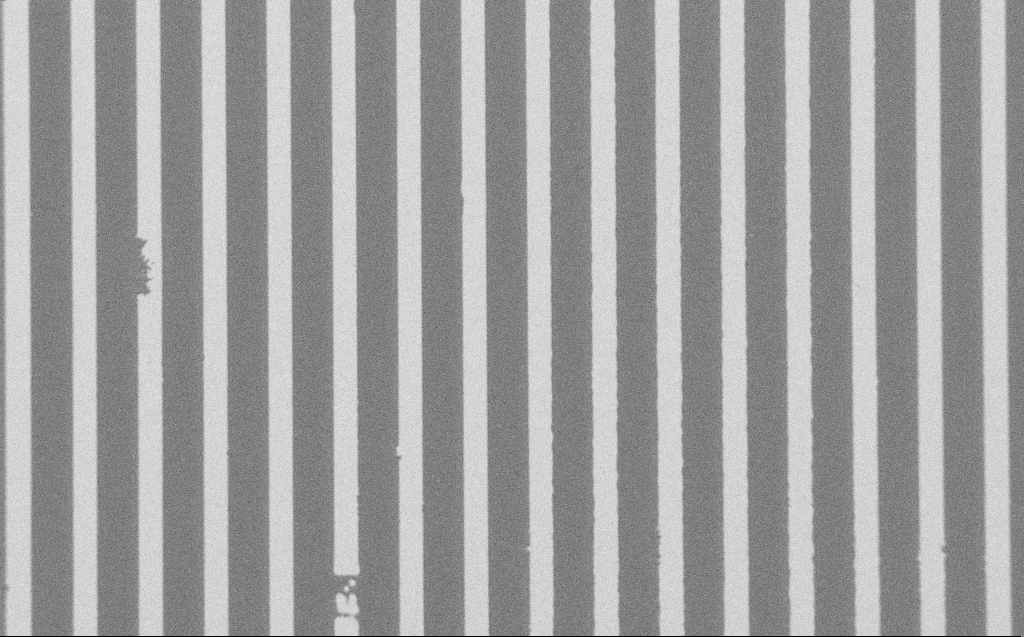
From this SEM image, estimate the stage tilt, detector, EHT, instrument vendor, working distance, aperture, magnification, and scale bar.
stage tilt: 0°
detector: SE2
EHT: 1.2 kV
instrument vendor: Zeiss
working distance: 6 mm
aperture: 30 µm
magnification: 0.856 K X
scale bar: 20000 nm